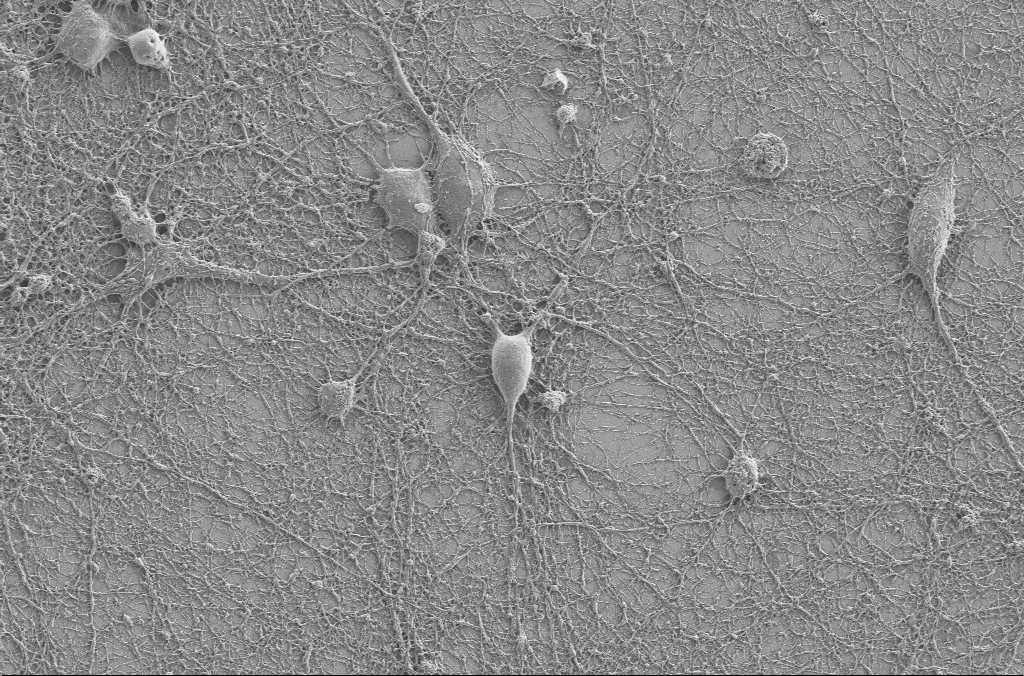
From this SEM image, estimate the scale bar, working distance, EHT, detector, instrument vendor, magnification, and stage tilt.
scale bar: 10000 nm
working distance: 4 mm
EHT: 2 kV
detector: SE2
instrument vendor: Zeiss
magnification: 2.5 K X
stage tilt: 0°